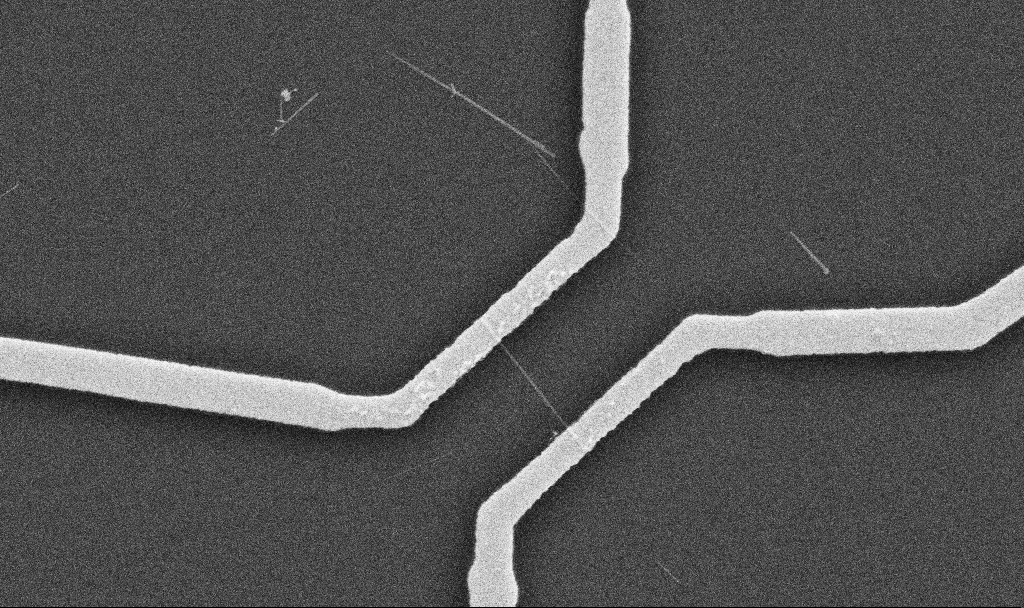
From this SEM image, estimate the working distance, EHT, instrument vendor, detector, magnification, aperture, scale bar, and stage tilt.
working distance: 10.7 mm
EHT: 10 kV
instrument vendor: Zeiss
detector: SE2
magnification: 20 K X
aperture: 30 µm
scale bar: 1000 nm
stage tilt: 0°